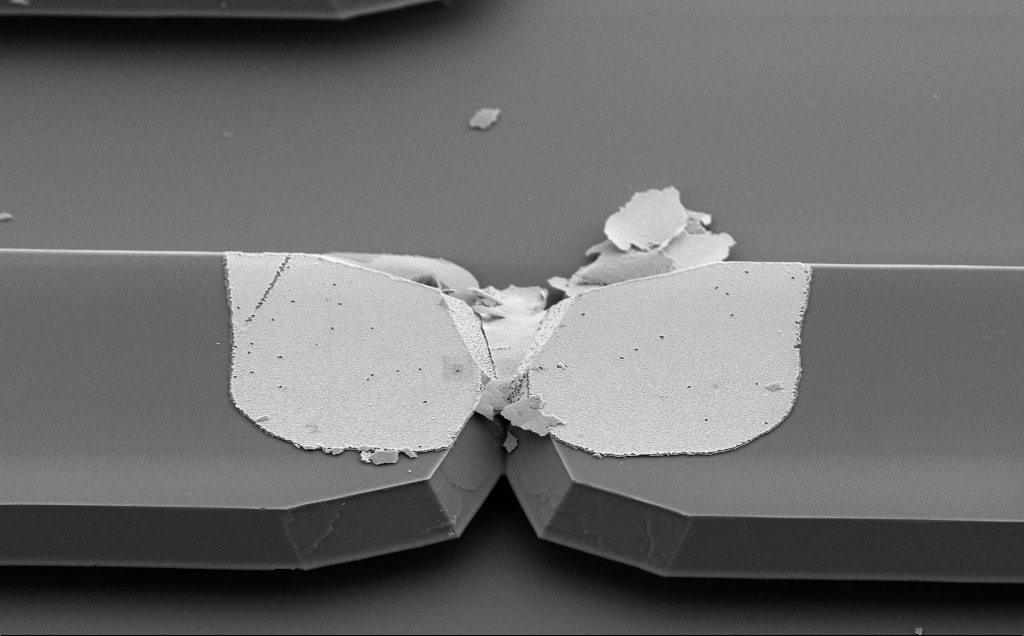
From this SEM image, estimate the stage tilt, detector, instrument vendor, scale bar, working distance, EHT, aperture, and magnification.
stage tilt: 50°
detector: SE2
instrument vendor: Zeiss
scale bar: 2000 nm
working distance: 10 mm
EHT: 5 kV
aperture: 30 µm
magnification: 8 K X